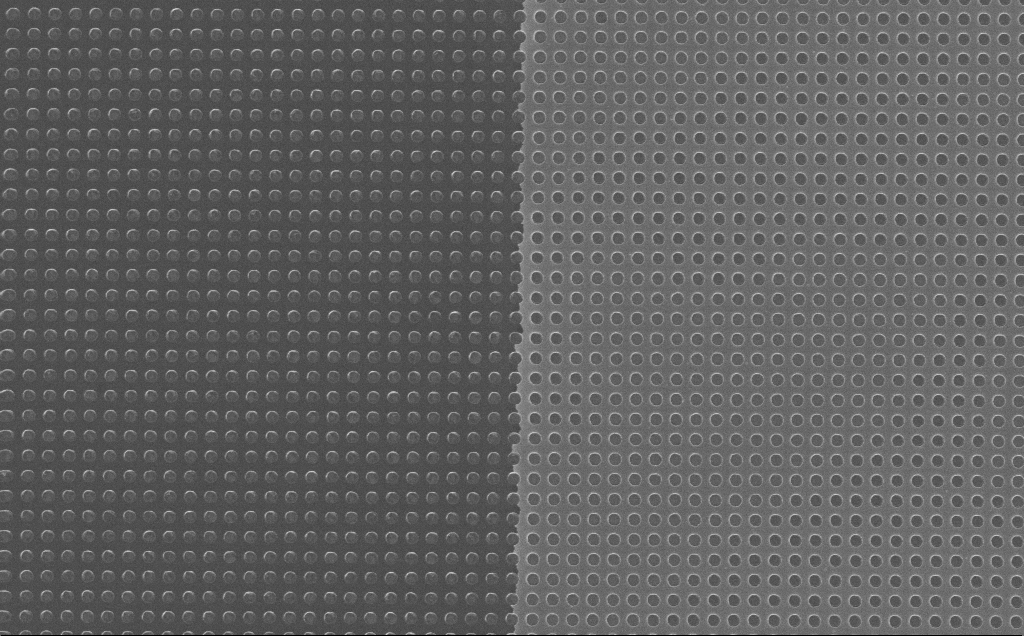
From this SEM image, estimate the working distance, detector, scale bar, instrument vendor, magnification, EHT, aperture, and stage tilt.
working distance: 7 mm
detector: InLens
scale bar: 2000 nm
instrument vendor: Zeiss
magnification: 18.58 K X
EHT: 10 kV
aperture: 30 µm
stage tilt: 0°